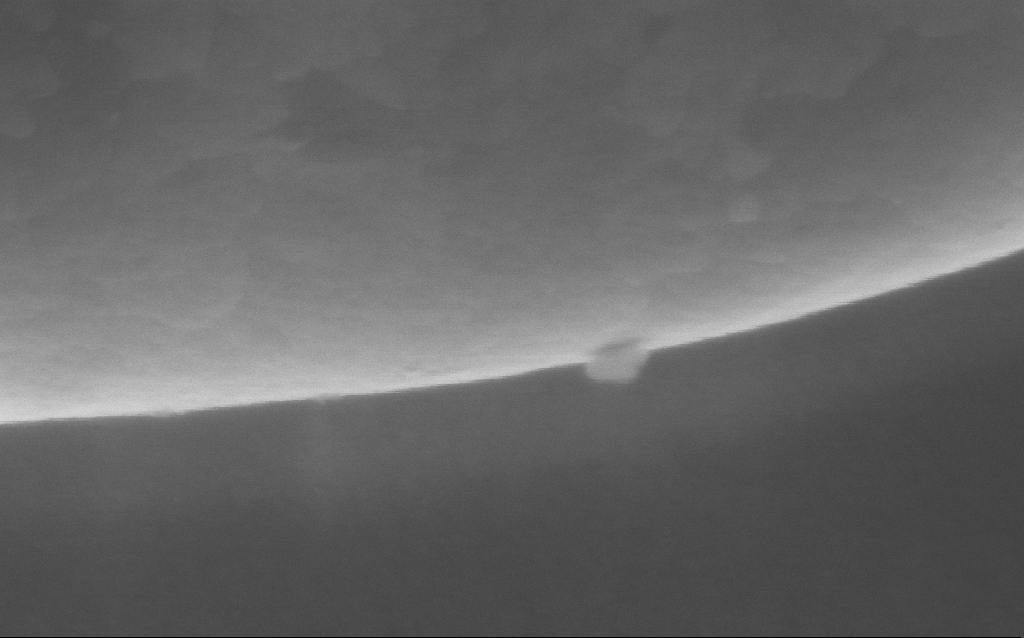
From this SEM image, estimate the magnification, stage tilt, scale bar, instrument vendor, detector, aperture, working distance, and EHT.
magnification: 298 K X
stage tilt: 0°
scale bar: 200 nm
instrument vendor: Zeiss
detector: InLens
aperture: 30 µm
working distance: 4 mm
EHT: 5 kV